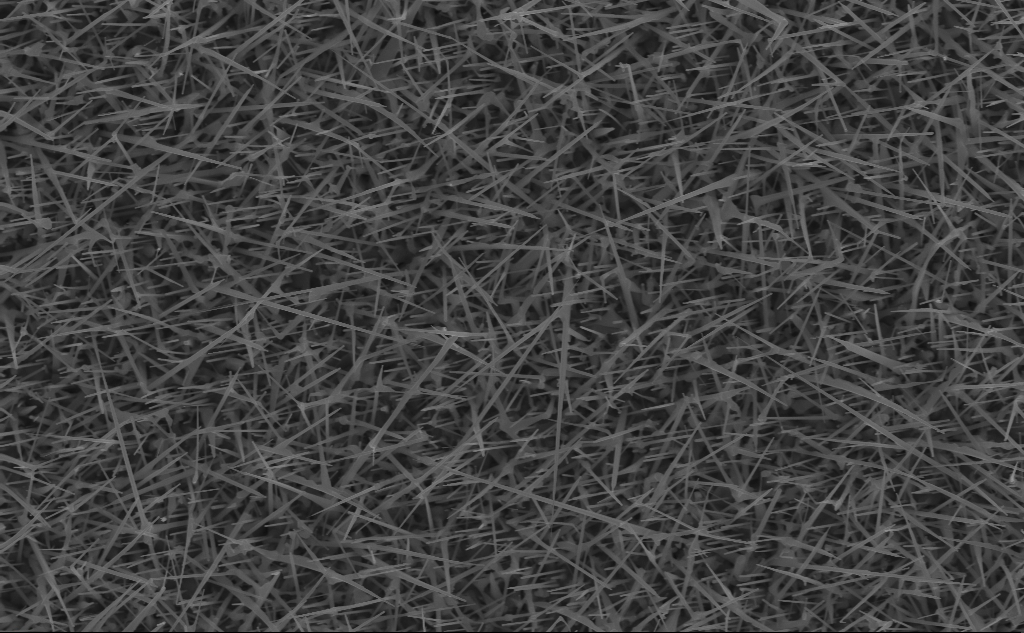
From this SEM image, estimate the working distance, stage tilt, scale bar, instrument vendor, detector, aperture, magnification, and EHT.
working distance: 7 mm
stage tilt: -0°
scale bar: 2000 nm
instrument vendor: Zeiss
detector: InLens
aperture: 30 µm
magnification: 20 K X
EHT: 10 kV